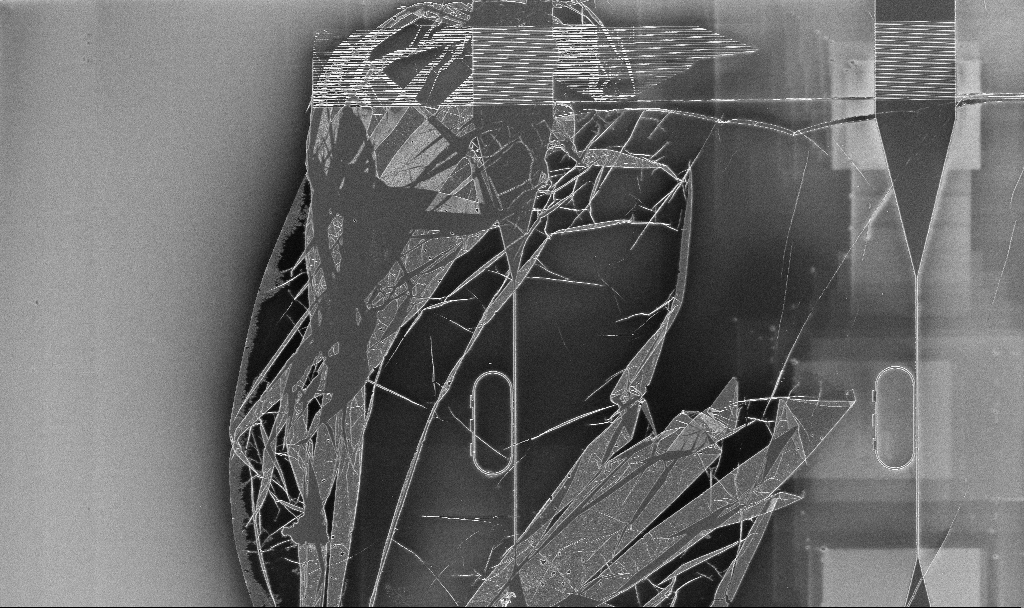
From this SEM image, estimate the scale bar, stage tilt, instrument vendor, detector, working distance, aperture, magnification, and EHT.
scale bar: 10000 nm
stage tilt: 0°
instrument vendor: Zeiss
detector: InLens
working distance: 5.2 mm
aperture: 30 µm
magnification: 1.49 K X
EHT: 5 kV